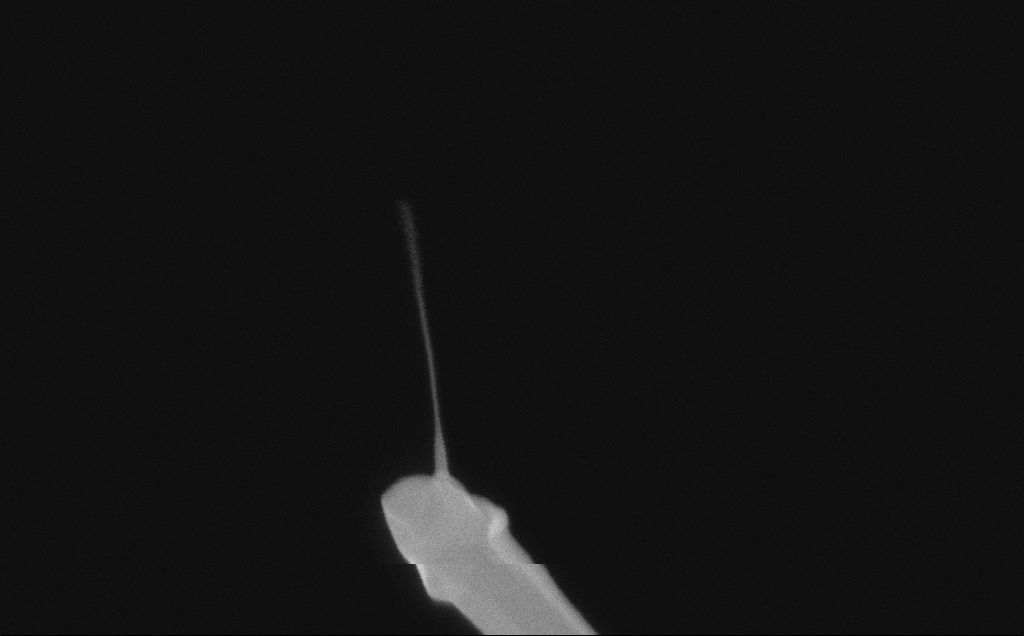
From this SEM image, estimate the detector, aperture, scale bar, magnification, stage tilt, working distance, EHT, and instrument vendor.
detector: InLens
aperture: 30 µm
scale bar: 200 nm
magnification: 189.64 K X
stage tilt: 0°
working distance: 6 mm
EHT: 10 kV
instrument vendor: Zeiss